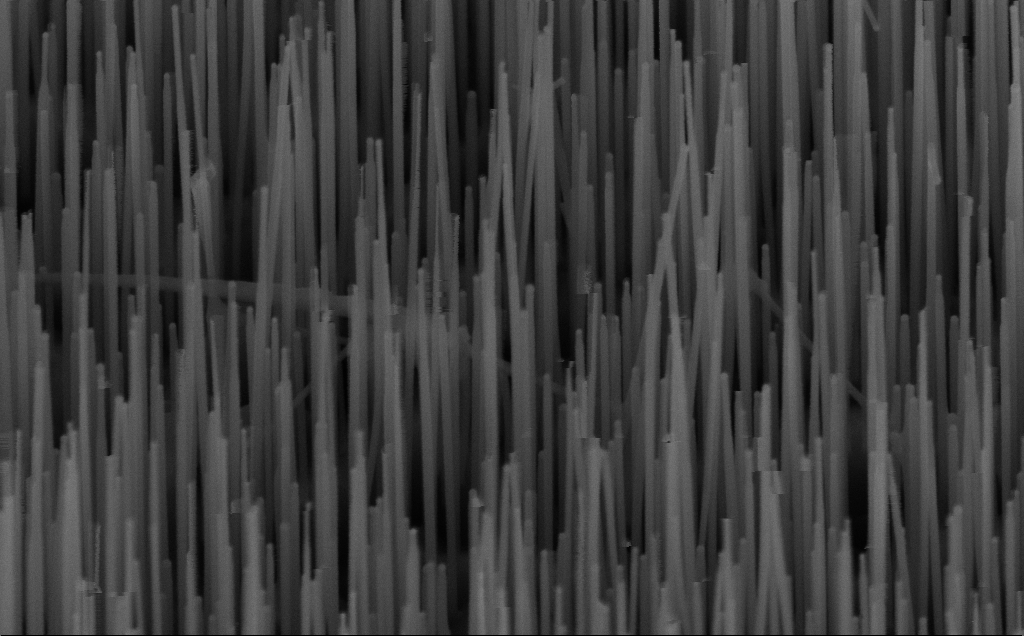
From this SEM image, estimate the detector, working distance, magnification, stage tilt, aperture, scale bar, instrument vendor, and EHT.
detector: InLens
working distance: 7 mm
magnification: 100 K X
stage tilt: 45°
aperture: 30 µm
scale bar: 200 nm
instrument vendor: Zeiss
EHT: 10 kV